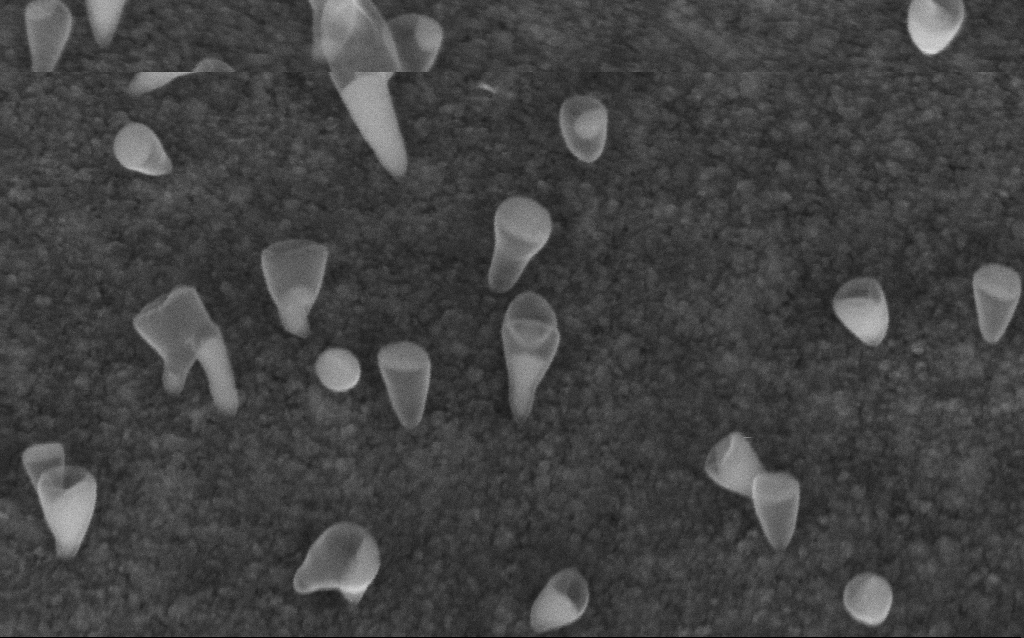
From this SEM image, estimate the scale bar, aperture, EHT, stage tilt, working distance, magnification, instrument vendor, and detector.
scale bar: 200 nm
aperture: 30 µm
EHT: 5 kV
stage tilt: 45°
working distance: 6.6 mm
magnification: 200 K X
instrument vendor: Zeiss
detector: InLens